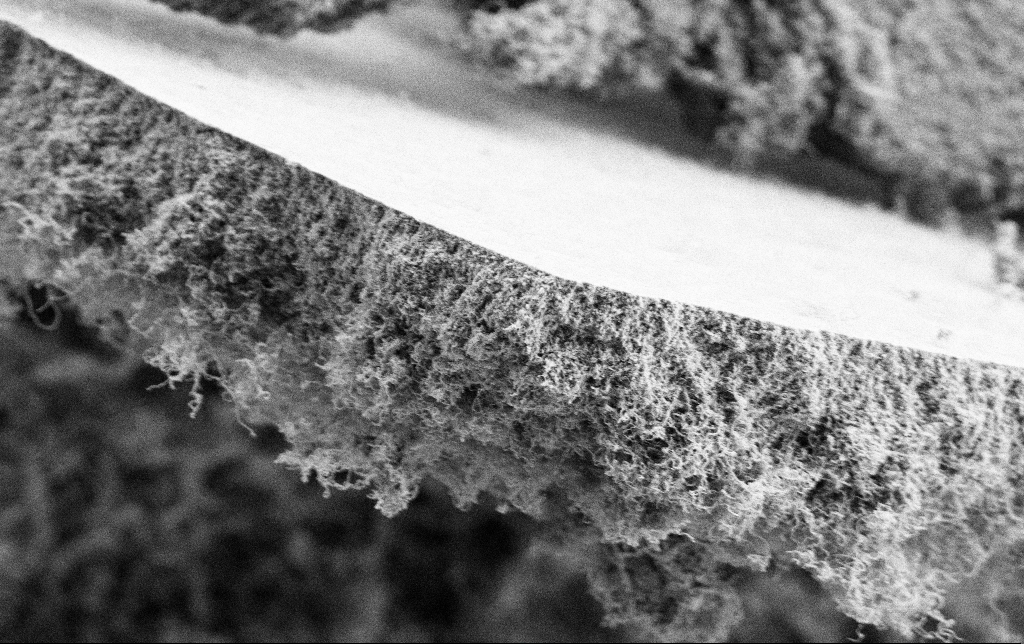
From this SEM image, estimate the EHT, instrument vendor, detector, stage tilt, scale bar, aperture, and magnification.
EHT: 2 kV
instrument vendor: Zeiss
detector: SE2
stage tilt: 0°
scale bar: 2000 nm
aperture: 30 µm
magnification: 10 K X